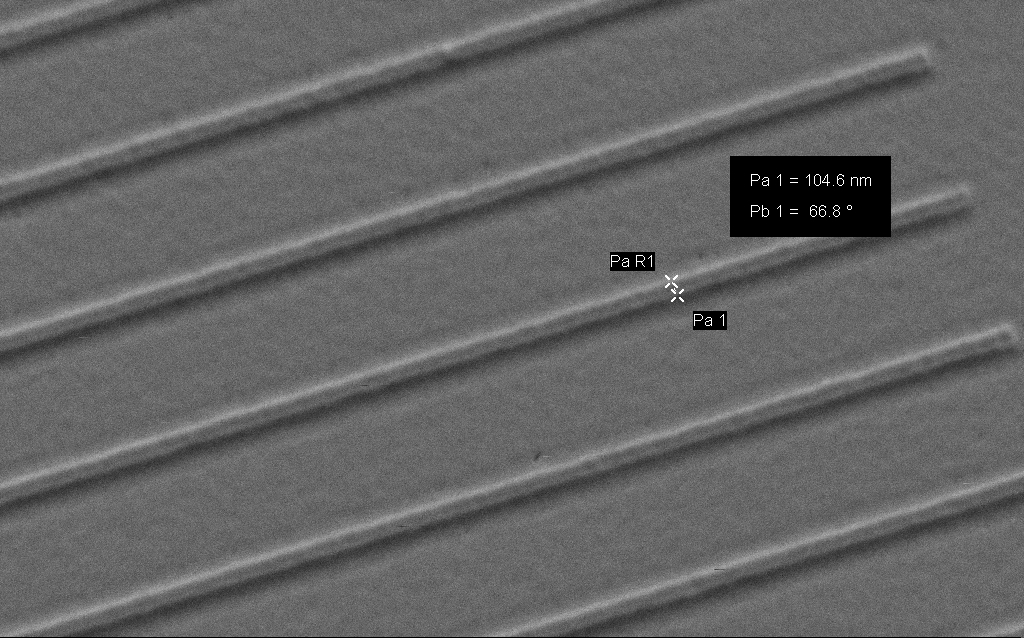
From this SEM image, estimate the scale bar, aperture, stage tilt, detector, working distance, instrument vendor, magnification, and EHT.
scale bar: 1000 nm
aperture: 30 µm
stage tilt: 45°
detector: SE2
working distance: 4 mm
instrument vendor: Zeiss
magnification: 53.48 K X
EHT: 2 kV